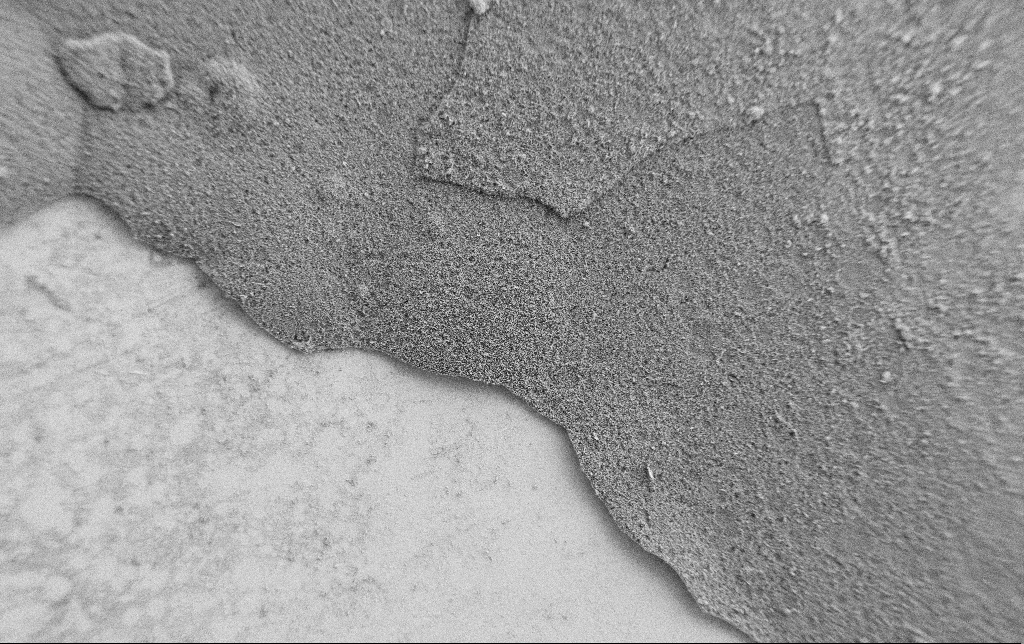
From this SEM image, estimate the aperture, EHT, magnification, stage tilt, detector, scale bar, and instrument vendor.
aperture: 30 µm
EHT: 2 kV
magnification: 0.15 K X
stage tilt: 0°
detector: SE2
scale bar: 100000 nm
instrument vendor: Zeiss